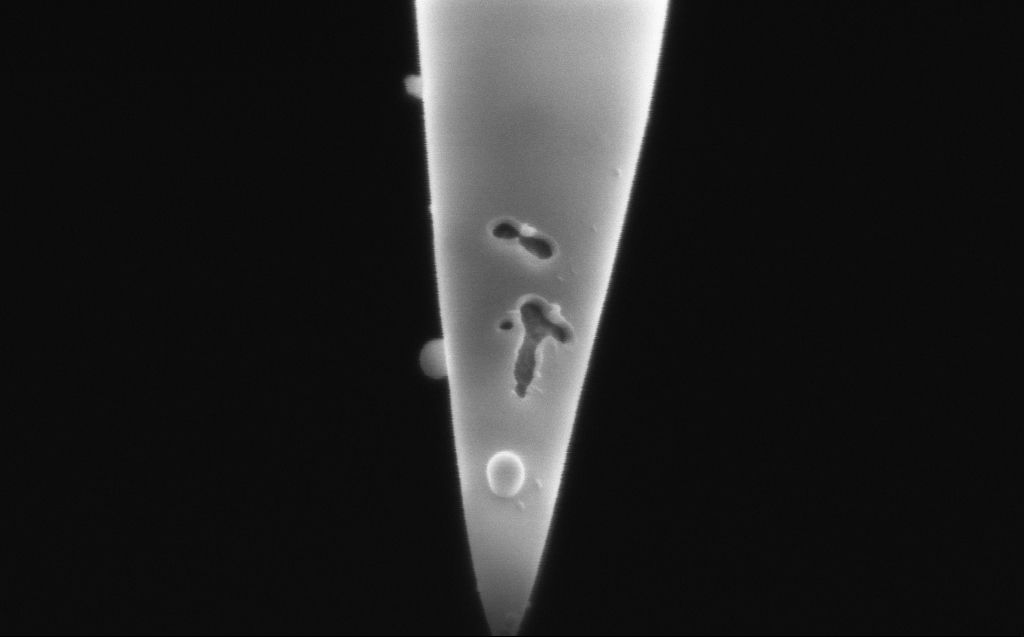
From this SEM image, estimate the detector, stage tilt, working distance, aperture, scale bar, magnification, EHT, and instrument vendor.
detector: InLens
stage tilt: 45.1°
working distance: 3 mm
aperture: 20 µm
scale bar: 200 nm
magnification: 131.69 K X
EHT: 2 kV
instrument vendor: Zeiss